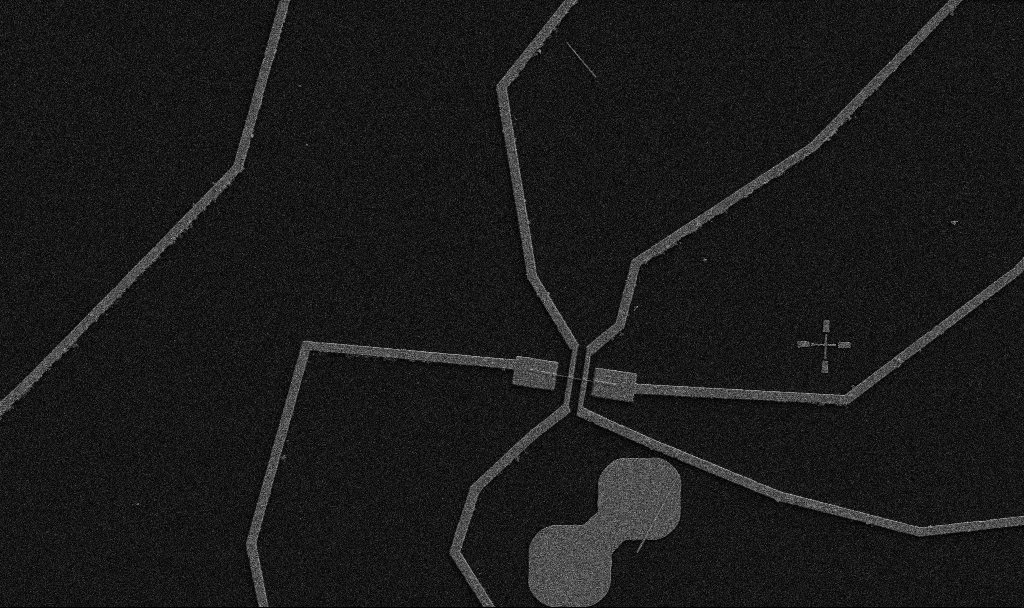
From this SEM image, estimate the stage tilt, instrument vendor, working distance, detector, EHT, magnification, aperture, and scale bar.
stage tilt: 0°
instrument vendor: Zeiss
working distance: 10.7 mm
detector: SE2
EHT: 5 kV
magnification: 5 K X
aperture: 30 µm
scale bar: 10000 nm